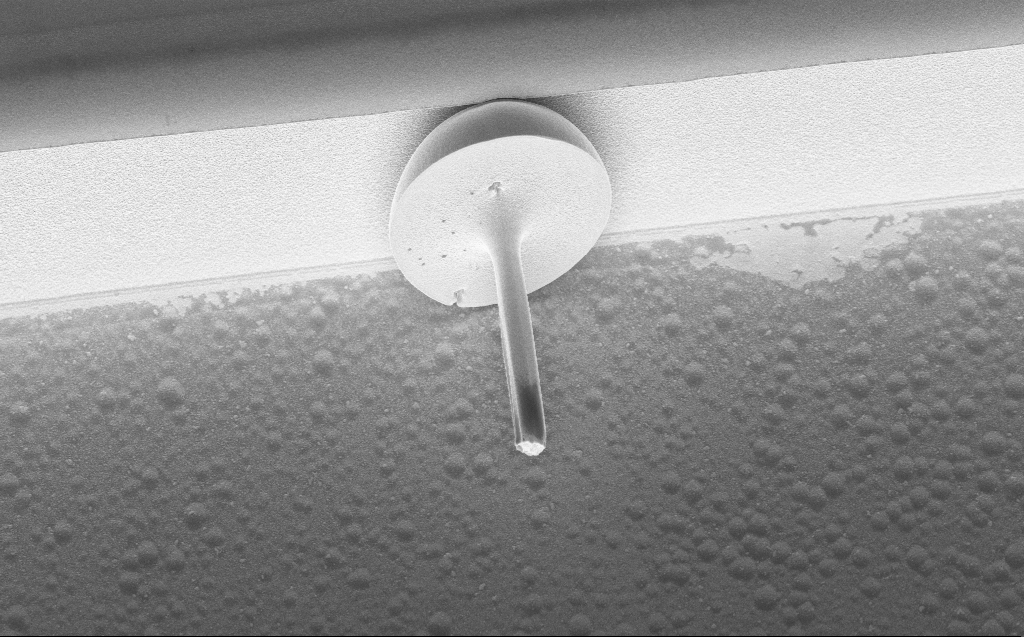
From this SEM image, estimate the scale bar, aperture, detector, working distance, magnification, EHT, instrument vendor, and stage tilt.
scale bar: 10000 nm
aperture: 30 µm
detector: InLens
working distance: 11 mm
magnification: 7.03 K X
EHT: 5 kV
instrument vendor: Zeiss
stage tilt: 44.4°